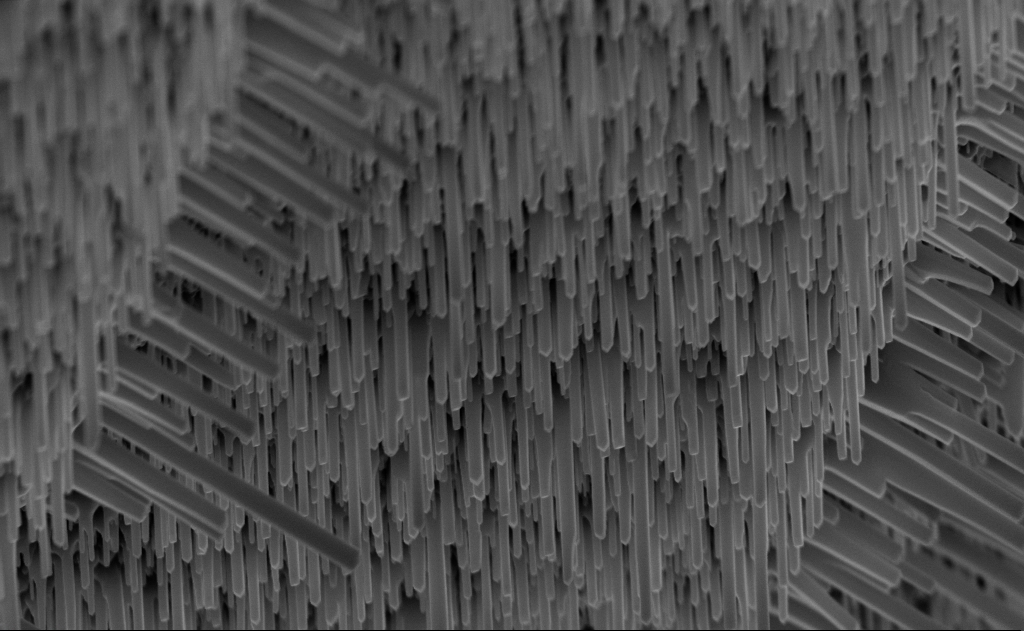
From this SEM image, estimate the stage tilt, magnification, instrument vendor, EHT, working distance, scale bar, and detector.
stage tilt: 0°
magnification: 40 K X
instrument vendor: Zeiss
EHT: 10 kV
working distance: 6 mm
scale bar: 1000 nm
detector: InLens